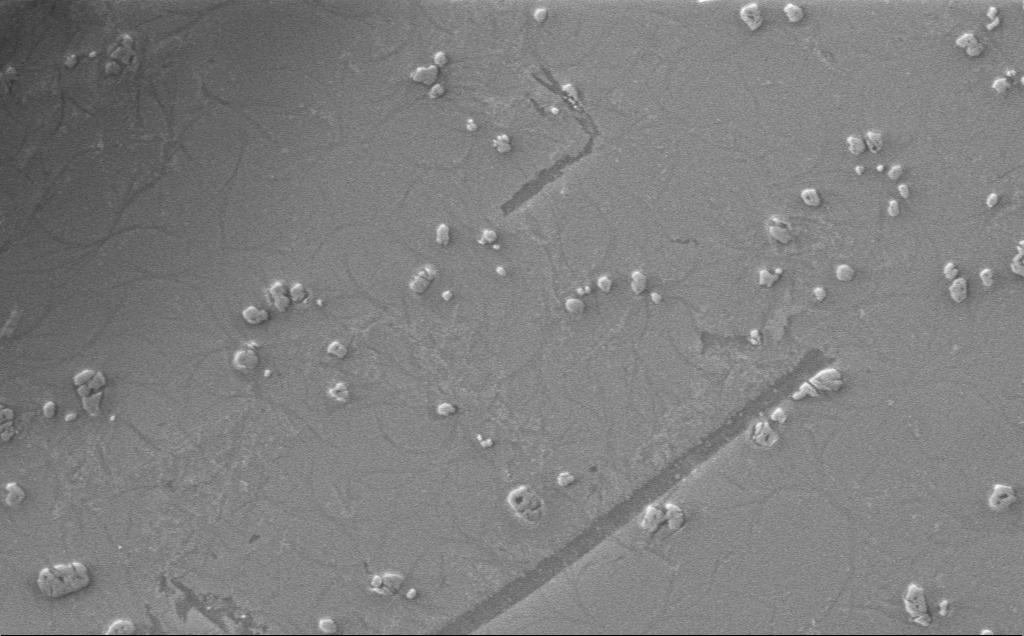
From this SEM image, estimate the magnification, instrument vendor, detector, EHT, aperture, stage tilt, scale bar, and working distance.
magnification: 6.26 K X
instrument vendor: Zeiss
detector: InLens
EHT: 1 kV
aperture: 30 µm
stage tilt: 0°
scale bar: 10000 nm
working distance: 4 mm